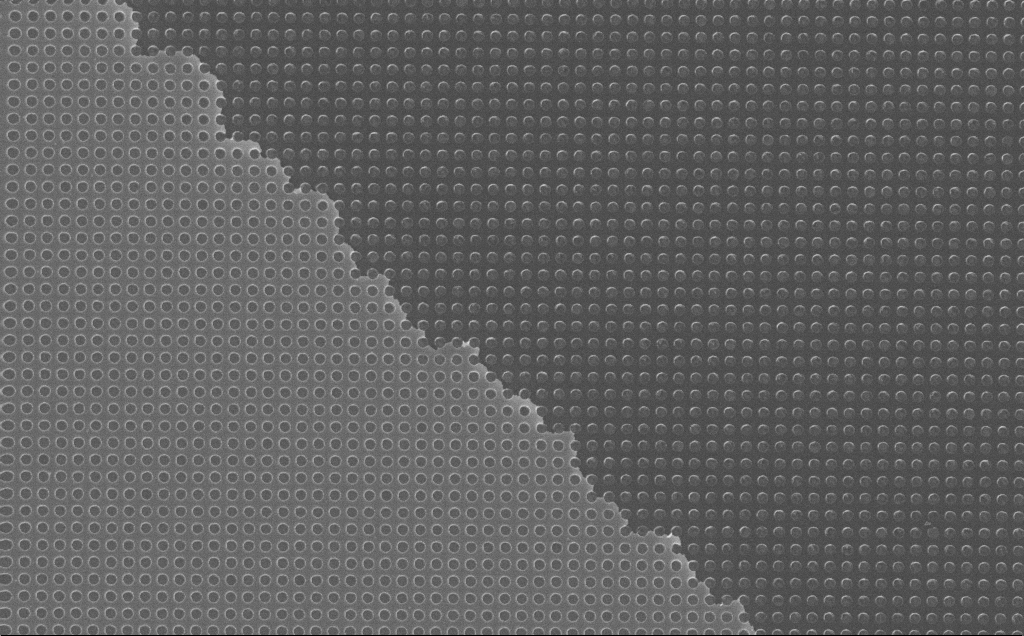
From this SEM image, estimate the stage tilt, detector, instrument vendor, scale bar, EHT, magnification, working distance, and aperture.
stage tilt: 0°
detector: InLens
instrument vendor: Zeiss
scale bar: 2000 nm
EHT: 10 kV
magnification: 18.01 K X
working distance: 7 mm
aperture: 30 µm